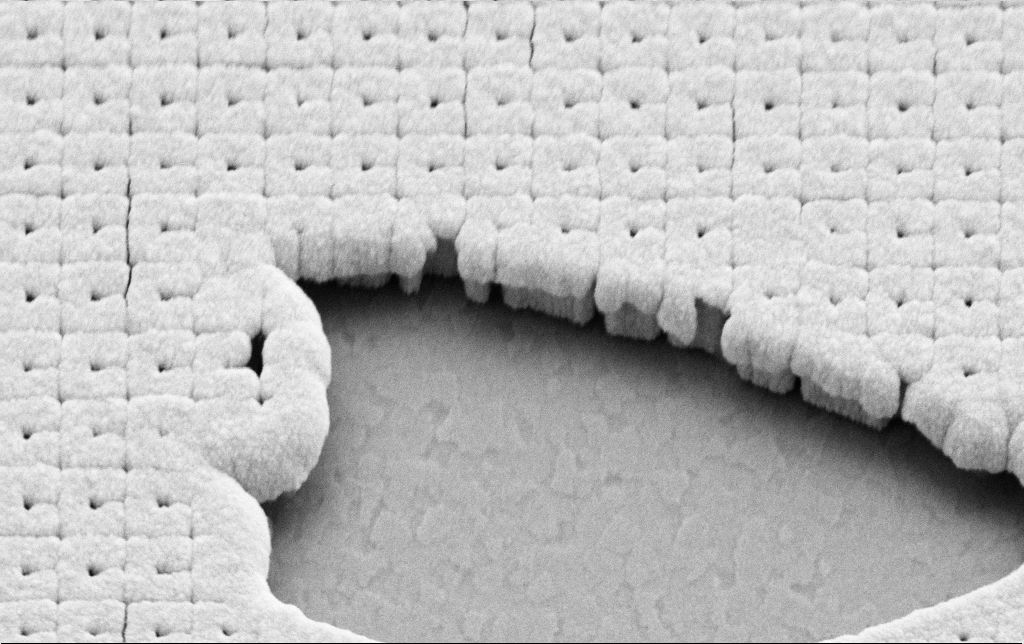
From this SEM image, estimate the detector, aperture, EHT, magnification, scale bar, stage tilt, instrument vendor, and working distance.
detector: SE2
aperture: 30 µm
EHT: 5 kV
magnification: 53.48 K X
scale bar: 1000 nm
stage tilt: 45°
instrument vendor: Zeiss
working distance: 6.6 mm